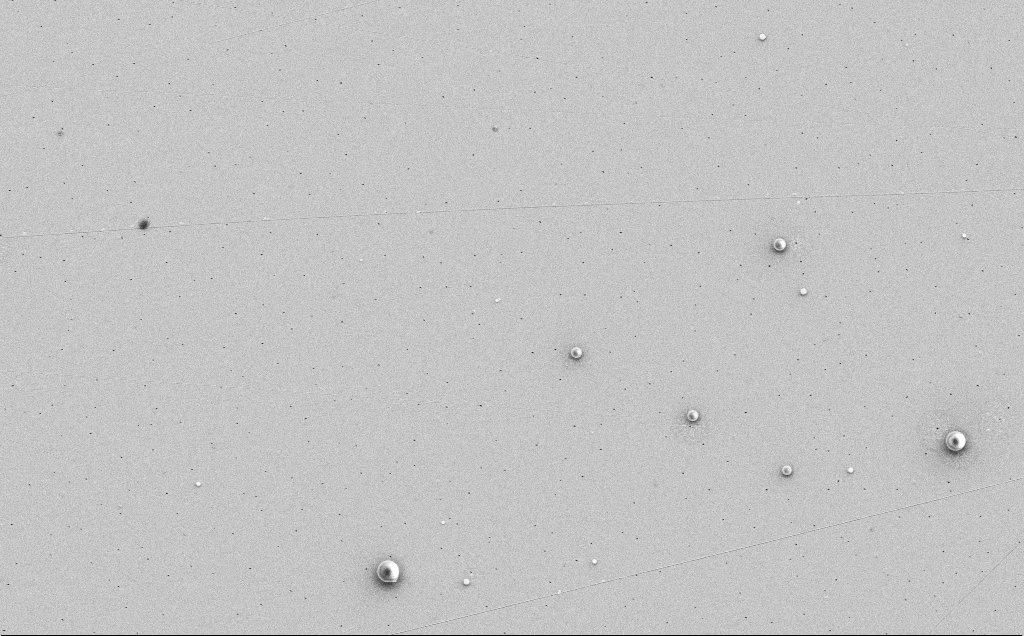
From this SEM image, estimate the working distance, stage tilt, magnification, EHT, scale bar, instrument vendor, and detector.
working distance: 12 mm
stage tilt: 0°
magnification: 4.2 K X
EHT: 5 kV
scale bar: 10000 nm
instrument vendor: Zeiss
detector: SE2